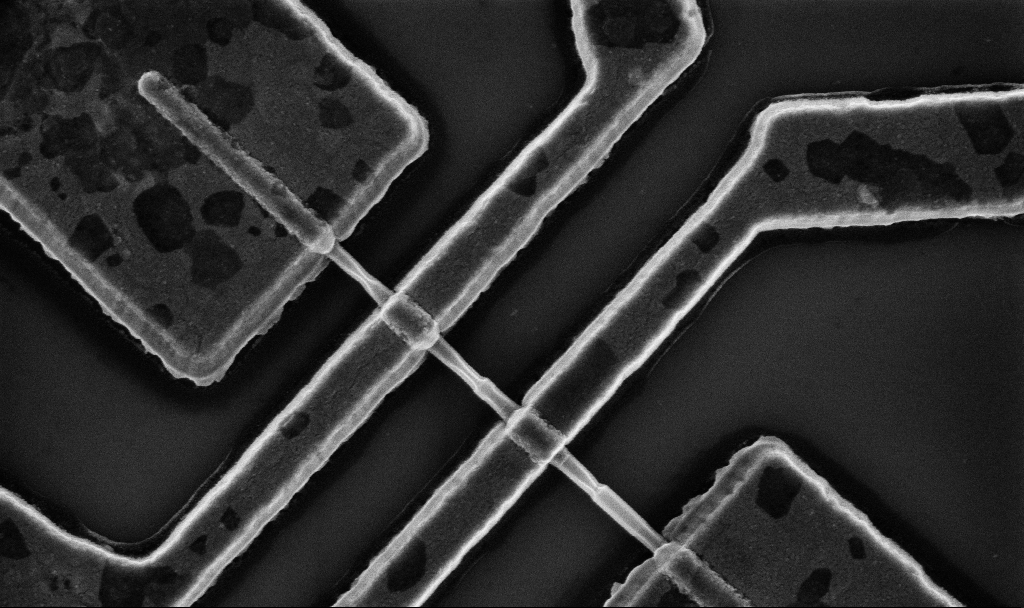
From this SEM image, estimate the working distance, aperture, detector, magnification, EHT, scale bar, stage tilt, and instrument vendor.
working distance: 10.7 mm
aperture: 30 µm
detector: InLens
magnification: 60 K X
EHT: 5 kV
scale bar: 1000 nm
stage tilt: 0°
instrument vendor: Zeiss